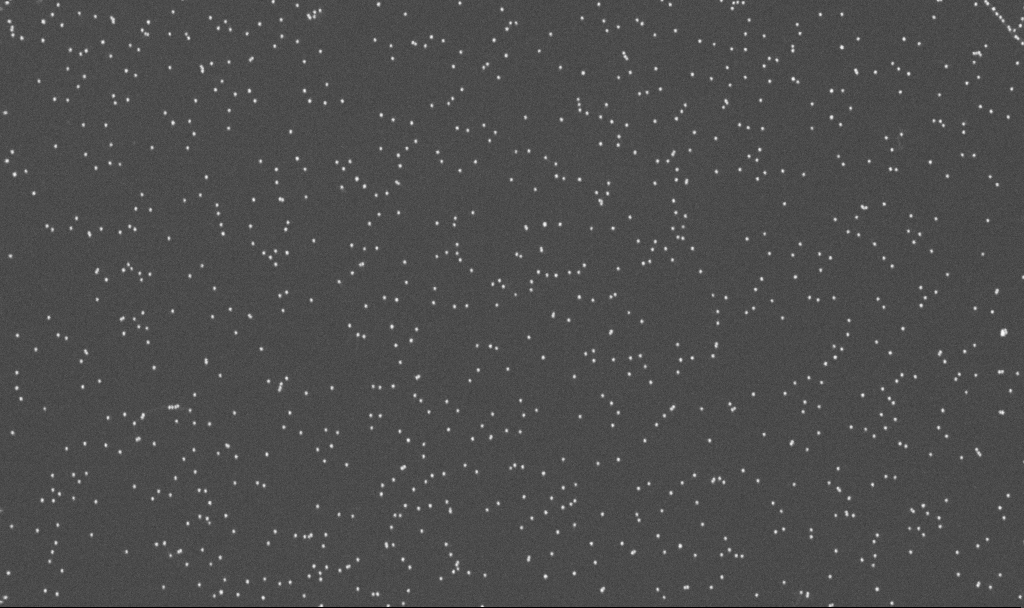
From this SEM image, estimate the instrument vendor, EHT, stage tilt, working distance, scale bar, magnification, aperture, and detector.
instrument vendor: Zeiss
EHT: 10 kV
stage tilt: -0°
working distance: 3.3 mm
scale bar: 200 nm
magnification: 100 K X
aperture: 30 µm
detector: InLens